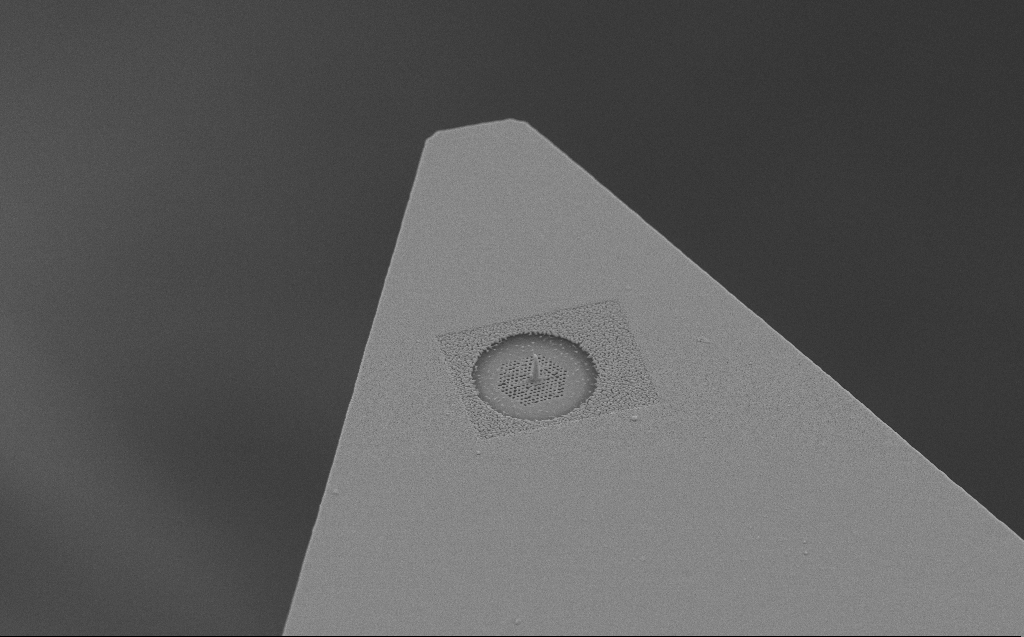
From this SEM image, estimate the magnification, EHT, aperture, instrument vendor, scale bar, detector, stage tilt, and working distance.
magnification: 6.45 K X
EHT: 10 kV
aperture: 30 µm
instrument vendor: Zeiss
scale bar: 10000 nm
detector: SE2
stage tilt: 45°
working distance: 6 mm